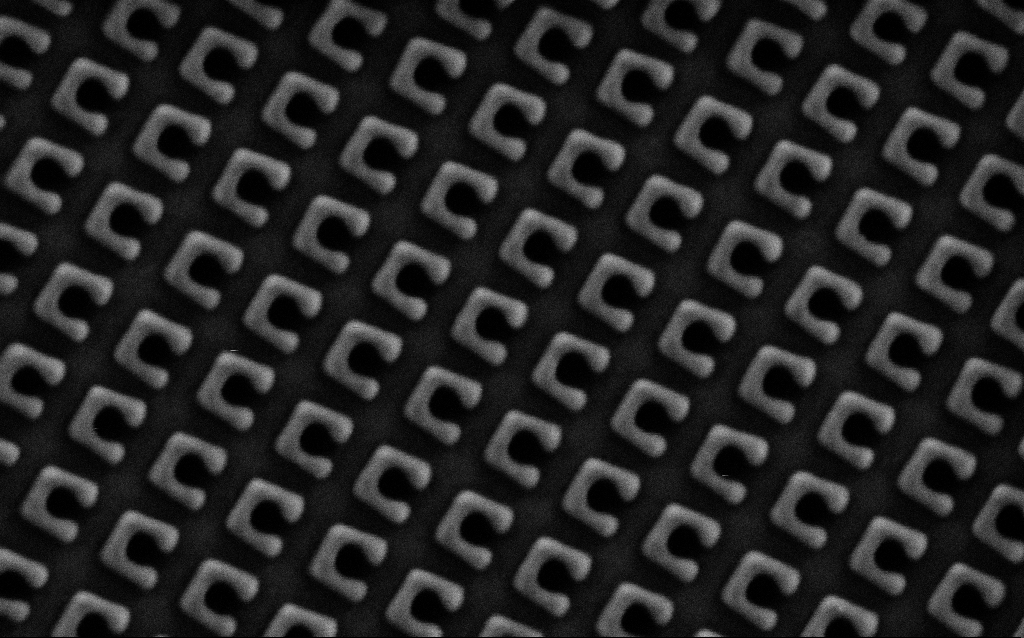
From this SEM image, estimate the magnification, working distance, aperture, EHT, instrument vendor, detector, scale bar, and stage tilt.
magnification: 73.22 K X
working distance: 8 mm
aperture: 30 µm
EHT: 1.5 kV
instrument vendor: Zeiss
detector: SE2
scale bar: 200 nm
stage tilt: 0°